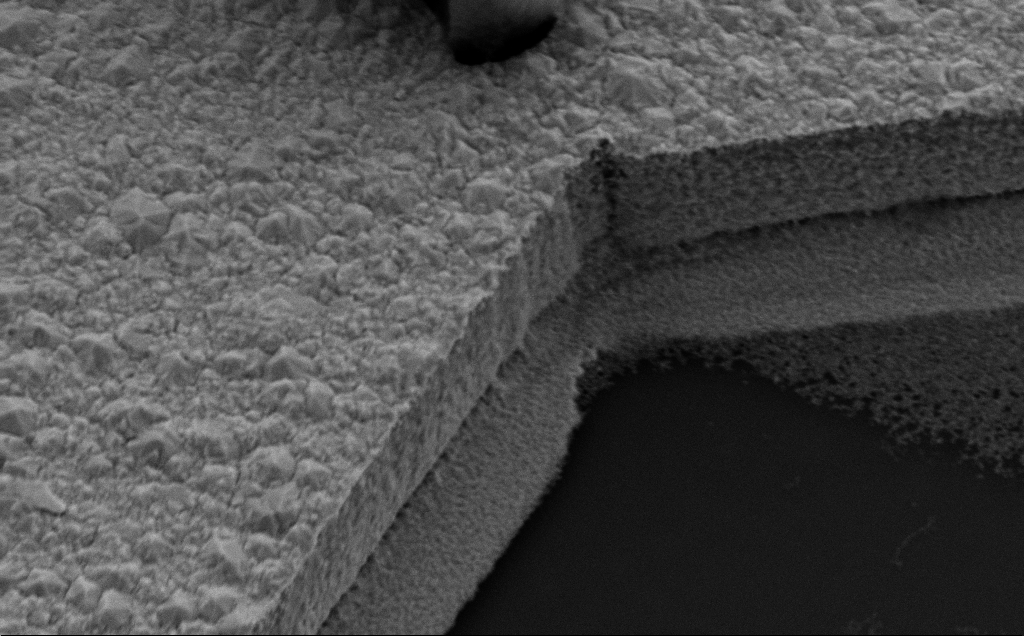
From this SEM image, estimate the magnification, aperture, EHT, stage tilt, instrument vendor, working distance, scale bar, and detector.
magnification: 17.02 K X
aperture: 30 µm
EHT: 10 kV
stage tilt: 35°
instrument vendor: Zeiss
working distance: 8 mm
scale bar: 2000 nm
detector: SE2